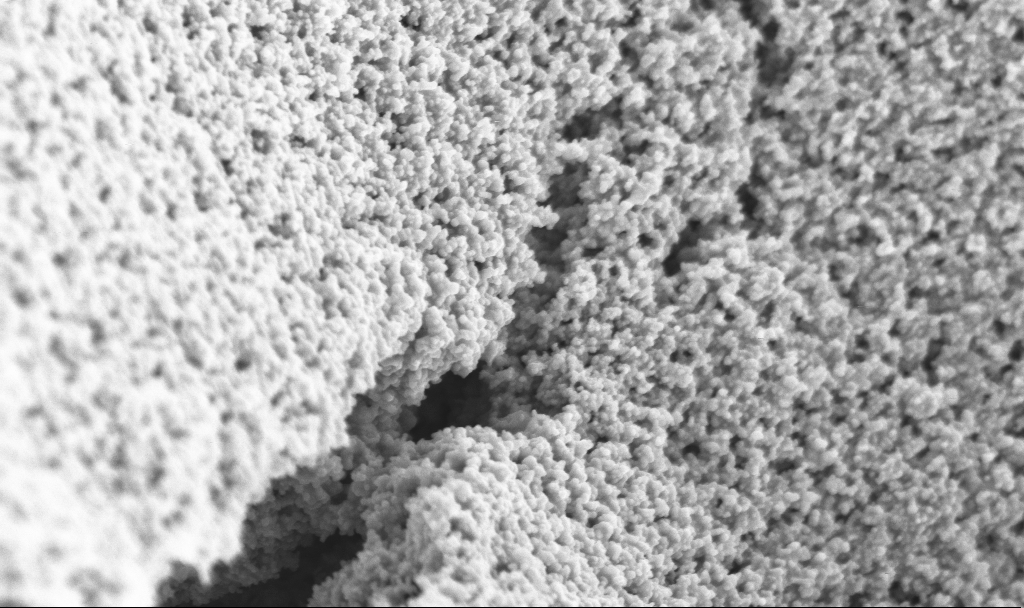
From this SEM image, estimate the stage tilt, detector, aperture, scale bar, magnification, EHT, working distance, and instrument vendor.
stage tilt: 0°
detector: InLens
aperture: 30 µm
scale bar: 1000 nm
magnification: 60.02 K X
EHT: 3 kV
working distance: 2.5 mm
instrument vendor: Zeiss